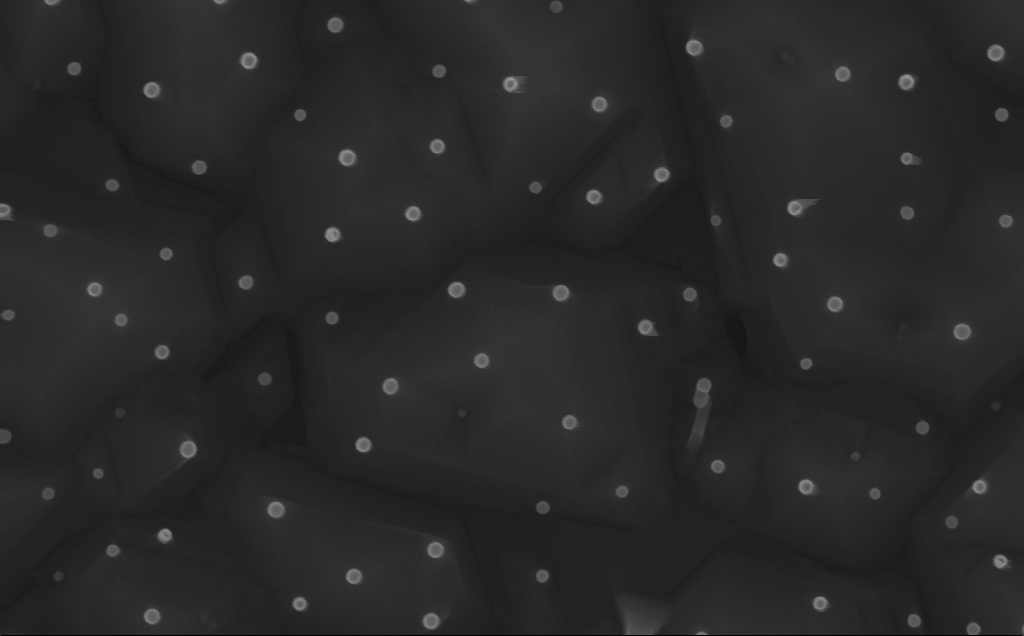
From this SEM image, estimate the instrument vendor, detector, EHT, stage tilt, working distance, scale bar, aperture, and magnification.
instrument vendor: Zeiss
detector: InLens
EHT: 10 kV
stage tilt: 0°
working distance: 6 mm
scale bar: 200 nm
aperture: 30 µm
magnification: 146.95 K X